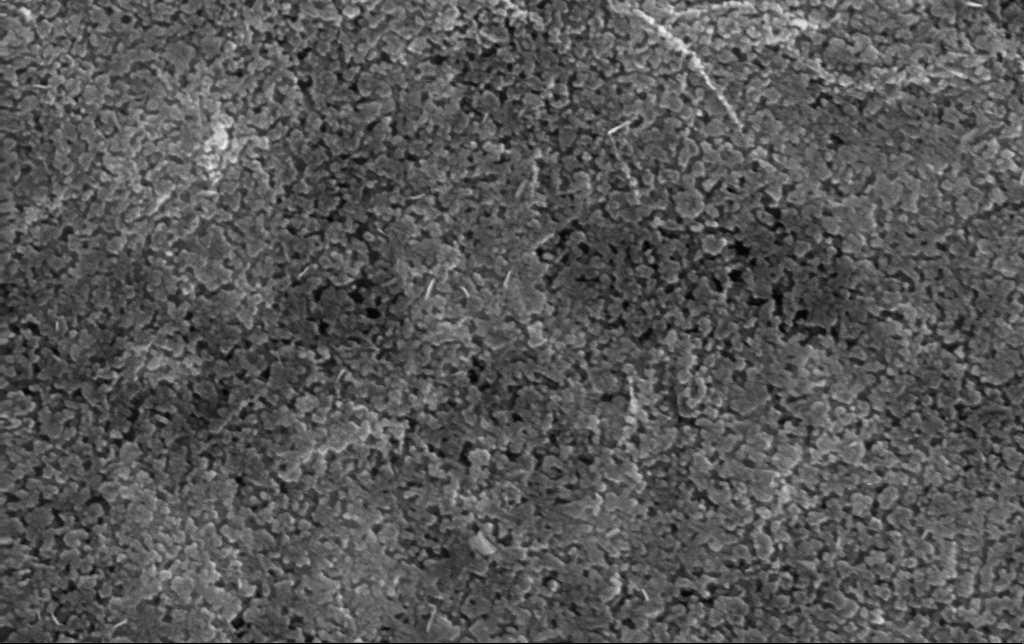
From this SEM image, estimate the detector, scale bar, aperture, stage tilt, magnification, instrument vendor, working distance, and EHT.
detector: InLens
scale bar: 100 nm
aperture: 30 µm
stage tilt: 0°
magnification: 150 K X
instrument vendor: Zeiss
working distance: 3 mm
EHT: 10 kV